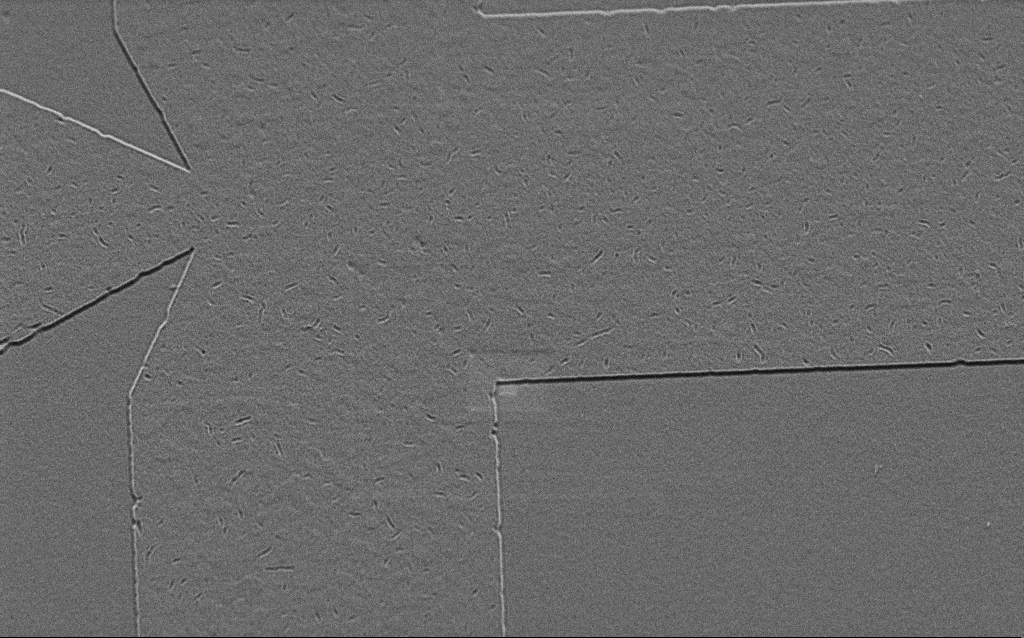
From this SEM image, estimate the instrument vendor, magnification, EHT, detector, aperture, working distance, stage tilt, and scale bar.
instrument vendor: Zeiss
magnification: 13.54 K X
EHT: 1.5 kV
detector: SE2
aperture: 30 µm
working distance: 6 mm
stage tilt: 45°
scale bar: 1000 nm